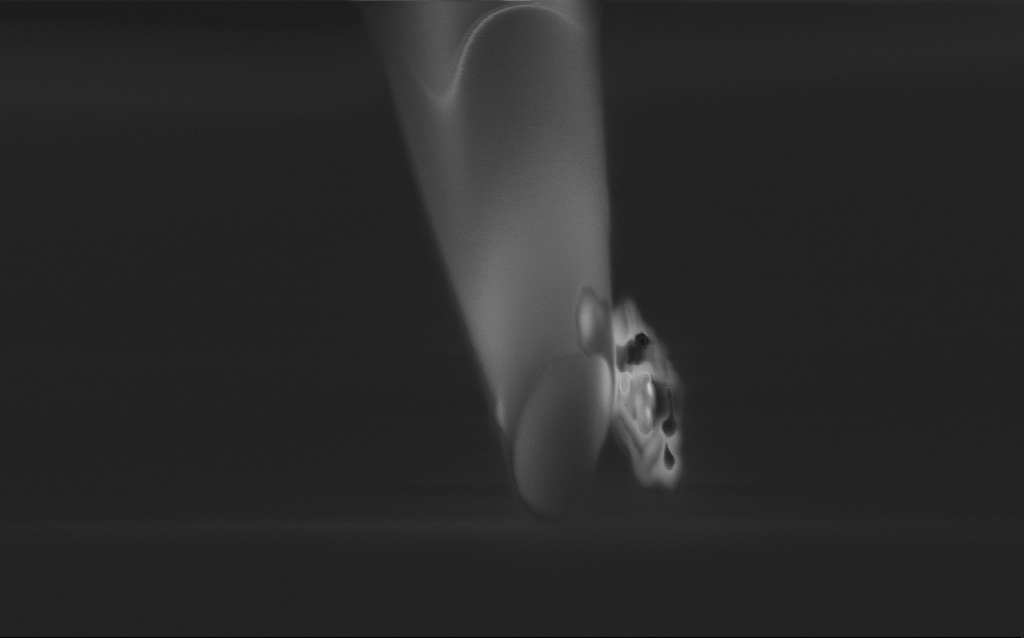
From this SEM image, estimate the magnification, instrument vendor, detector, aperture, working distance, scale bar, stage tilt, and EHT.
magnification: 65.84 K X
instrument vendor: Zeiss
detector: InLens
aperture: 30 µm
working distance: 5 mm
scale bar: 1000 nm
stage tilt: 45°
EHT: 2 kV